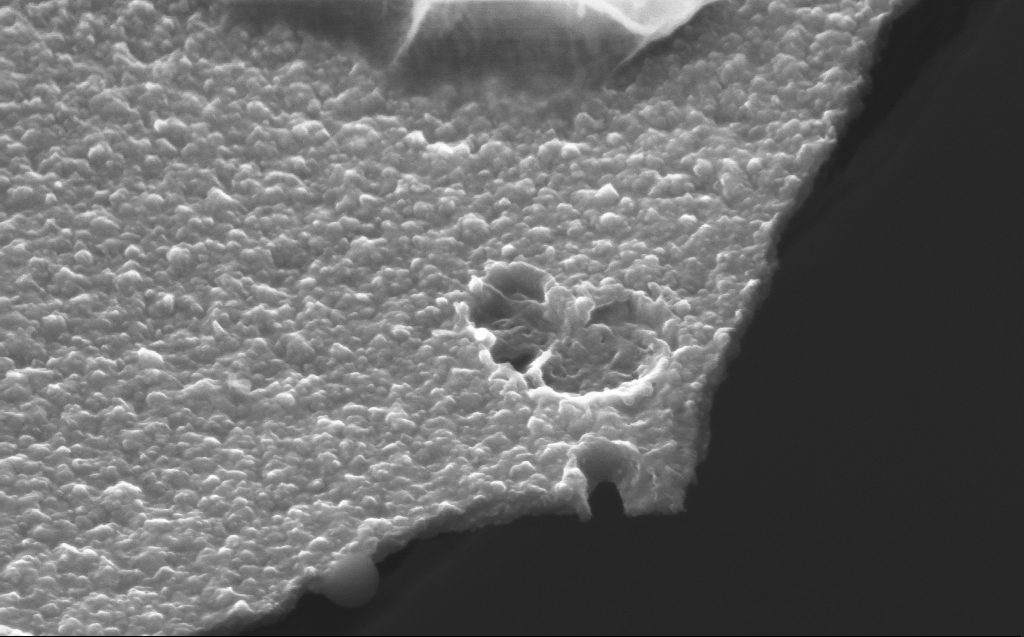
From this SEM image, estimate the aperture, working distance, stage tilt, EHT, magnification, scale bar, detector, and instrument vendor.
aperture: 30 µm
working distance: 4 mm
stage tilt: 45°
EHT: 5 kV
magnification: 105.5 K X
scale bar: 200 nm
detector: InLens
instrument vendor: Zeiss